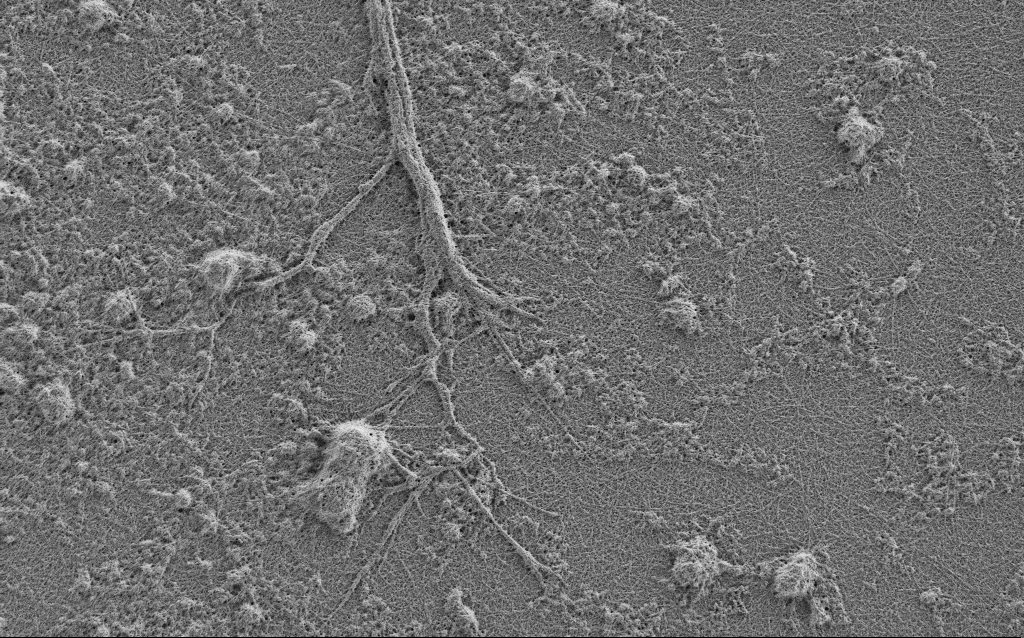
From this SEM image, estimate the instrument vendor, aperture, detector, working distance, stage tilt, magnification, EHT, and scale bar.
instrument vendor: Zeiss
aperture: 30 µm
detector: SE2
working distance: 4 mm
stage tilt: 0°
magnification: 7.5 K X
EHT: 0.9 kV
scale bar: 2000 nm